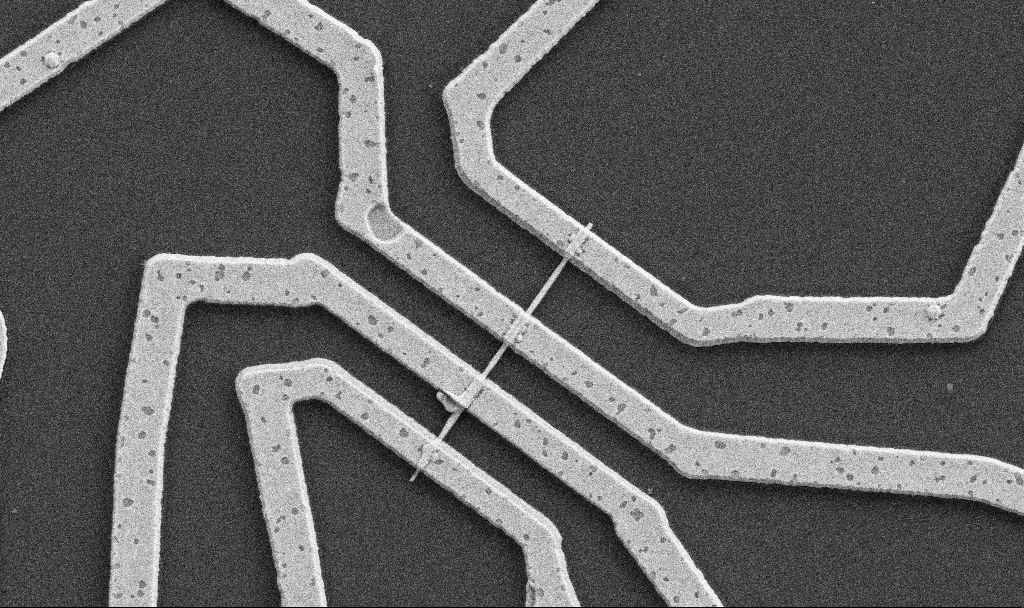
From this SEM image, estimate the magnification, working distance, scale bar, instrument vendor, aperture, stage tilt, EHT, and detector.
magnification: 20 K X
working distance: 10.7 mm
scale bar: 1000 nm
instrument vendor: Zeiss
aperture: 30 µm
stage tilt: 0°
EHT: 5 kV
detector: SE2